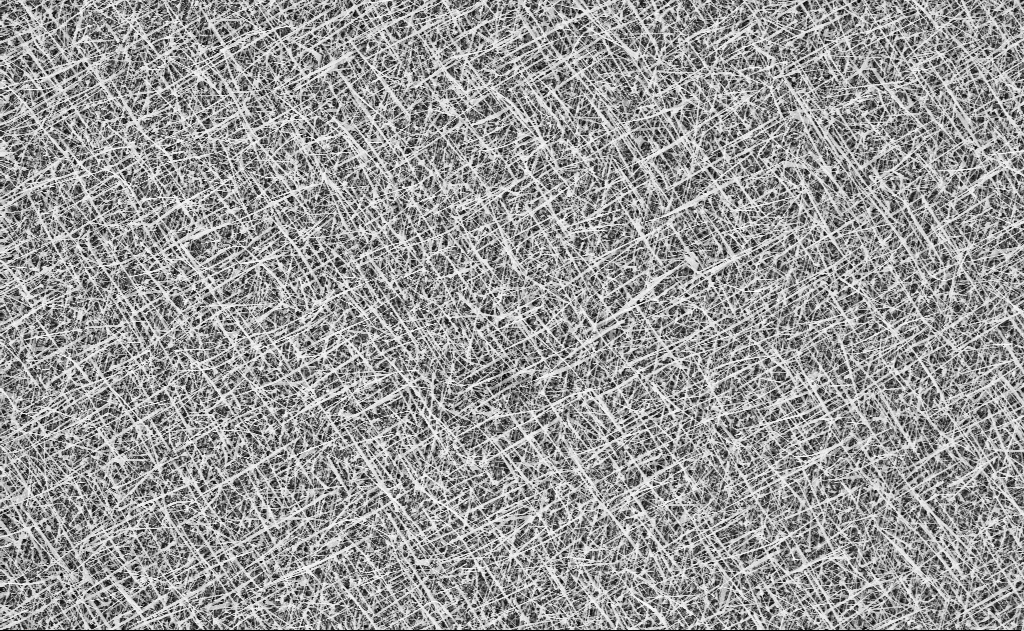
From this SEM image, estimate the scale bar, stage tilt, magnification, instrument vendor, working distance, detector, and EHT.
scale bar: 10000 nm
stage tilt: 0°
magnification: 5 K X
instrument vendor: Zeiss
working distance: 20 mm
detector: InLens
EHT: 10 kV